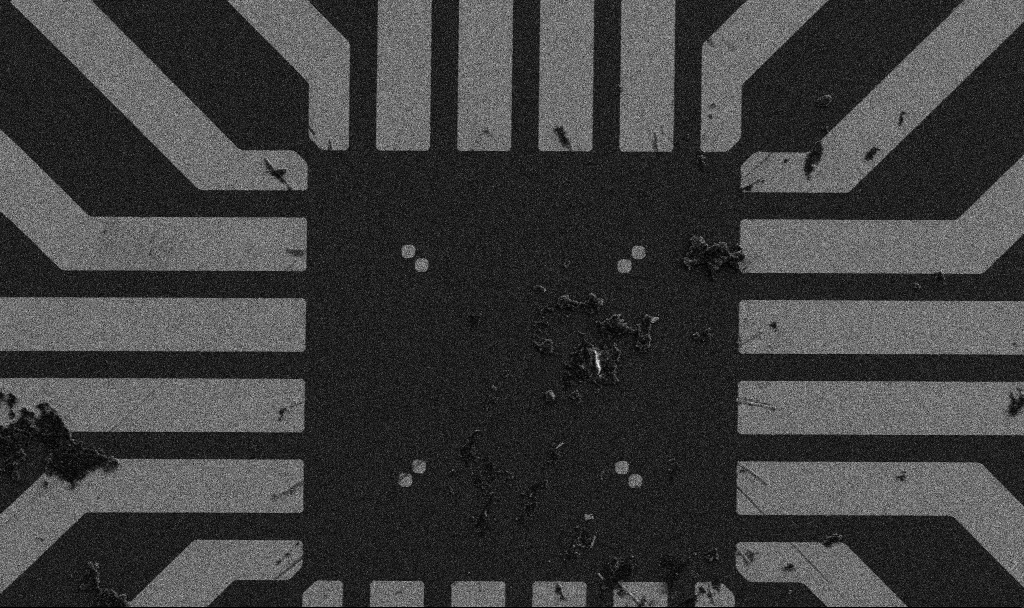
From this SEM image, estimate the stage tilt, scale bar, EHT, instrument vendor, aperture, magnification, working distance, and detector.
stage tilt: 0°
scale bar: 20000 nm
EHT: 5 kV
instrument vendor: Zeiss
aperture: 30 µm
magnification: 1 K X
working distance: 8.9 mm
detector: SE2